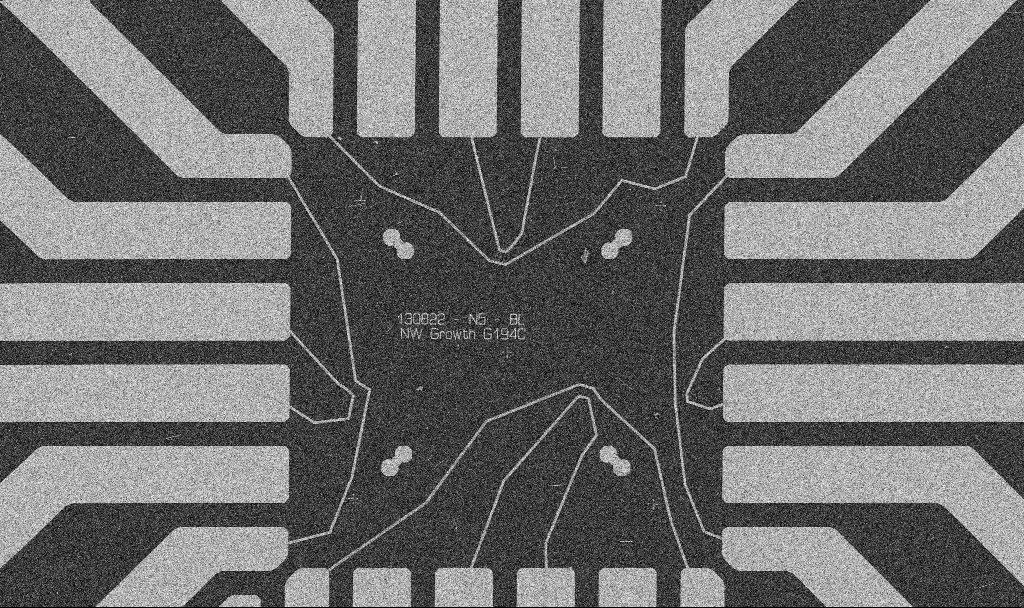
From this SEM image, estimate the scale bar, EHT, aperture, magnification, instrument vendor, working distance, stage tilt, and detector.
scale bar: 20000 nm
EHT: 5 kV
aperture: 30 µm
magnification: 1 K X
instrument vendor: Zeiss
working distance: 8.7 mm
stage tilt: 0°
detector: SE2